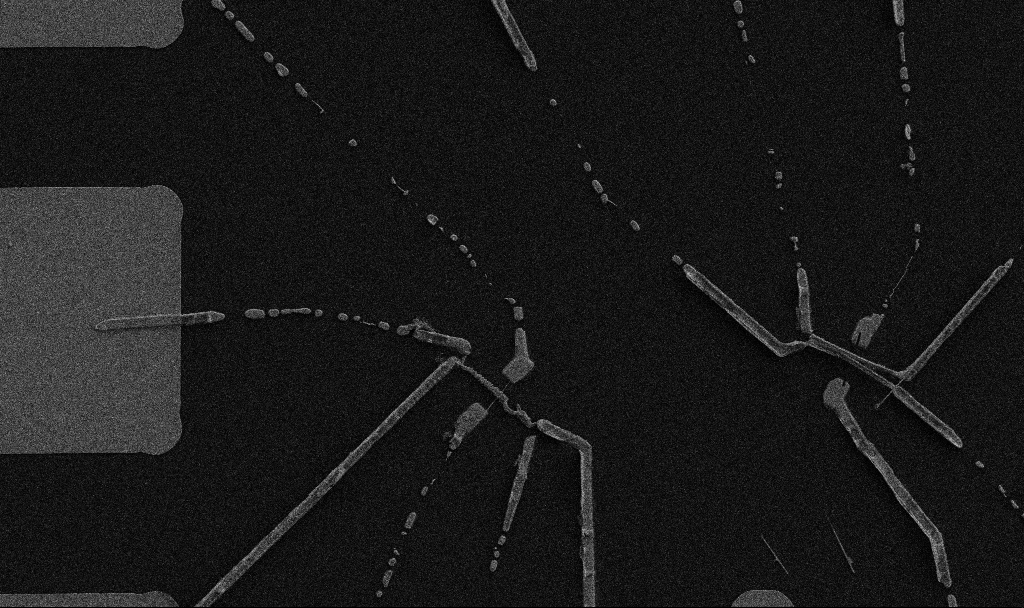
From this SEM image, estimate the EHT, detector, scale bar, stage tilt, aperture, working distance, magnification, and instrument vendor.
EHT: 5 kV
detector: SE2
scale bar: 10000 nm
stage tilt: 0°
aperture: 30 µm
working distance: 10.7 mm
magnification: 5 K X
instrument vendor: Zeiss